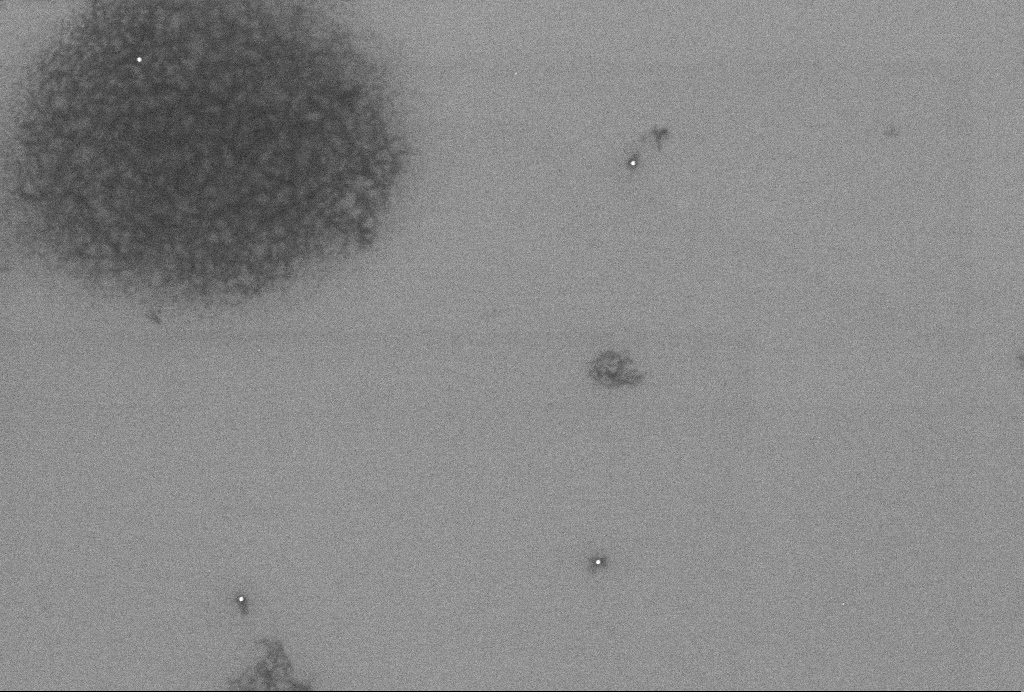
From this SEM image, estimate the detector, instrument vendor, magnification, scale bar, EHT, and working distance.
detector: InLens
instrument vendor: Zeiss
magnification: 55.95 K X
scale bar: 1000 nm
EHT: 2 kV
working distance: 3.3 mm